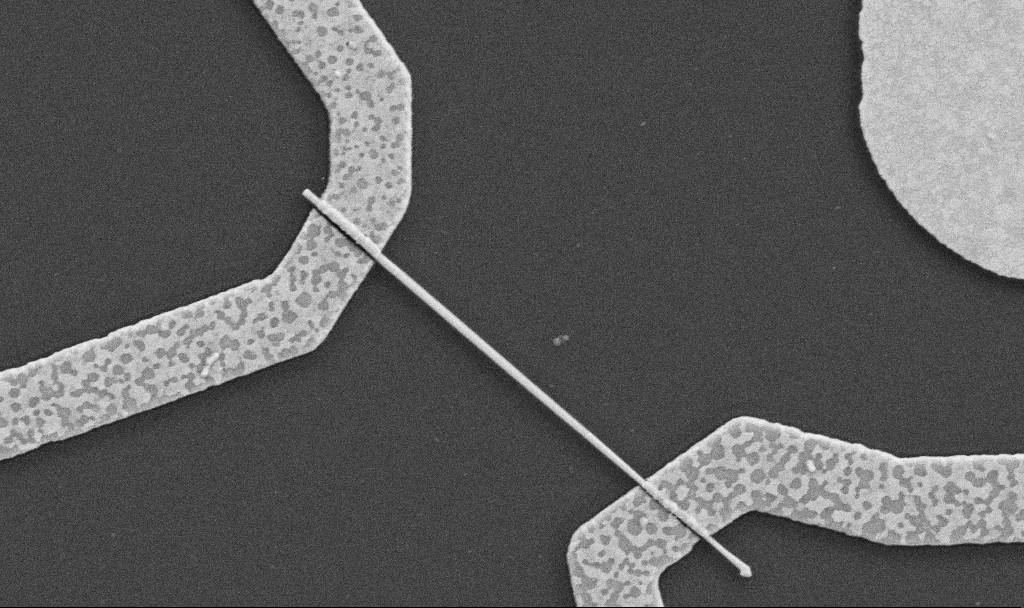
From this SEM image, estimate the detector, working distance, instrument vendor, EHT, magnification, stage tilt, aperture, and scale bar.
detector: SE2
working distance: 8.7 mm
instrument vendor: Zeiss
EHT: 5 kV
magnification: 30 K X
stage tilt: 0°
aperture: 30 µm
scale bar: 1000 nm